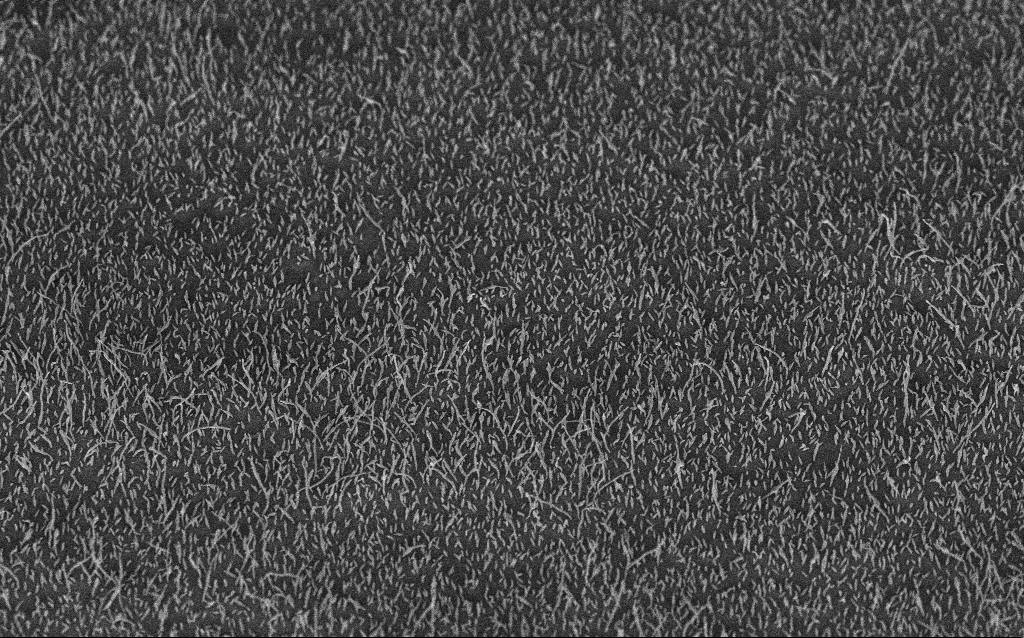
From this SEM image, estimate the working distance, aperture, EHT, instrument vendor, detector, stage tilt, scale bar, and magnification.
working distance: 6.4 mm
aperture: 30 µm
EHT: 5 kV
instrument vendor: Zeiss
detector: InLens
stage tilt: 45°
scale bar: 2000 nm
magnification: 10 K X